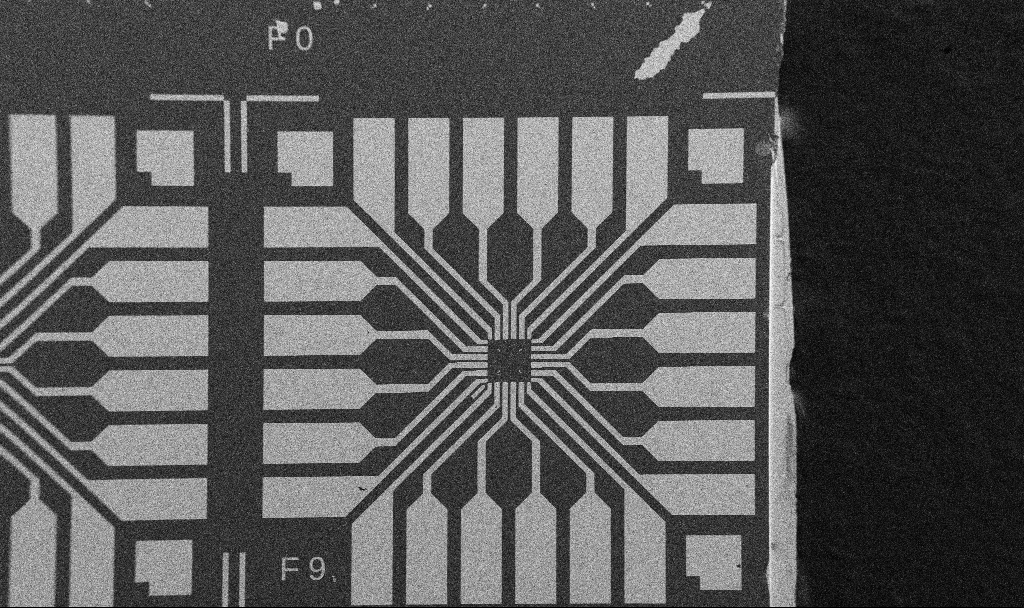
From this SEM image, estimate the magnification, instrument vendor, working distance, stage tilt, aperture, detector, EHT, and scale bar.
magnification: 0.1 K X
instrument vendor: Zeiss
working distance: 10.7 mm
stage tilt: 0°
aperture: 30 µm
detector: SE2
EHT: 5 kV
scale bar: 200000 nm